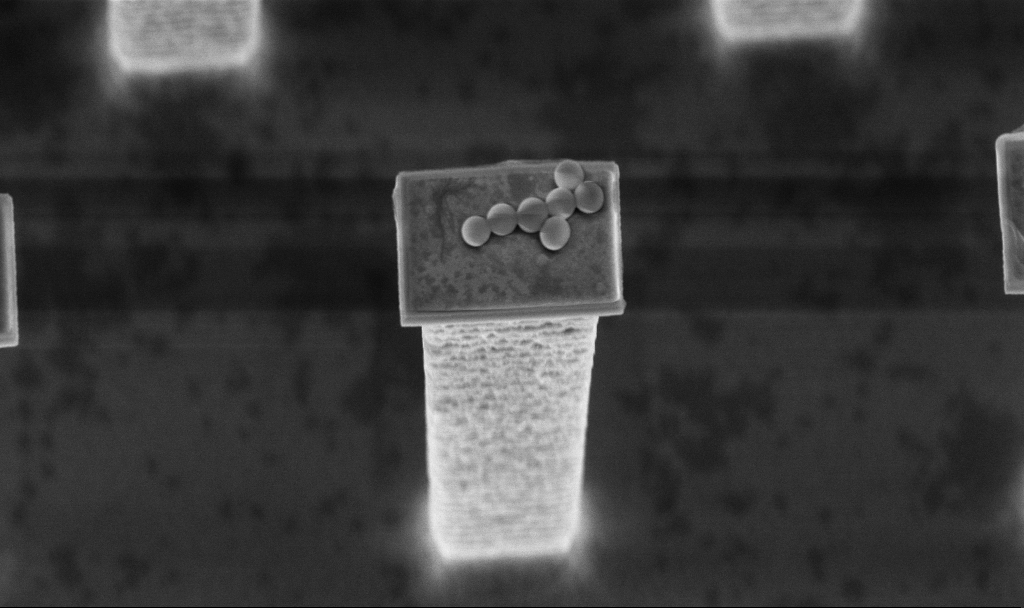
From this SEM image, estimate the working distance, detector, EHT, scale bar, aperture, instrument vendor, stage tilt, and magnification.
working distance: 3.2 mm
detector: InLens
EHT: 5 kV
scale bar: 1000 nm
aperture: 30 µm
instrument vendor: Zeiss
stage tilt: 20°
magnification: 18.42 K X